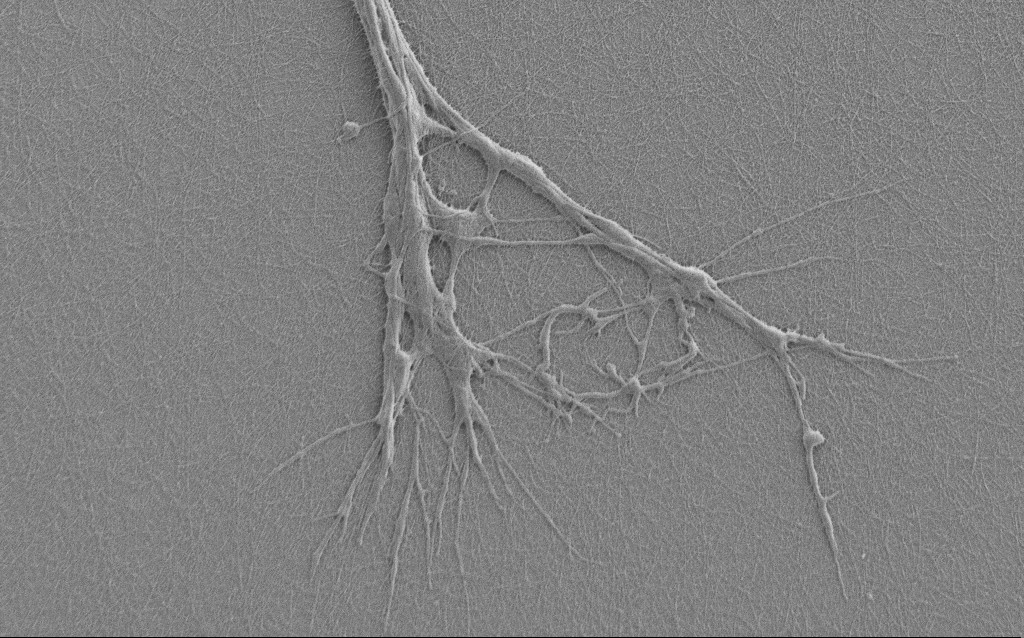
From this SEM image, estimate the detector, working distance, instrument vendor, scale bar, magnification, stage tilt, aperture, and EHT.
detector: SE2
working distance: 6 mm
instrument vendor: Zeiss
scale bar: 2000 nm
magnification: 7.5 K X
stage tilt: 0°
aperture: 30 µm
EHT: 1 kV